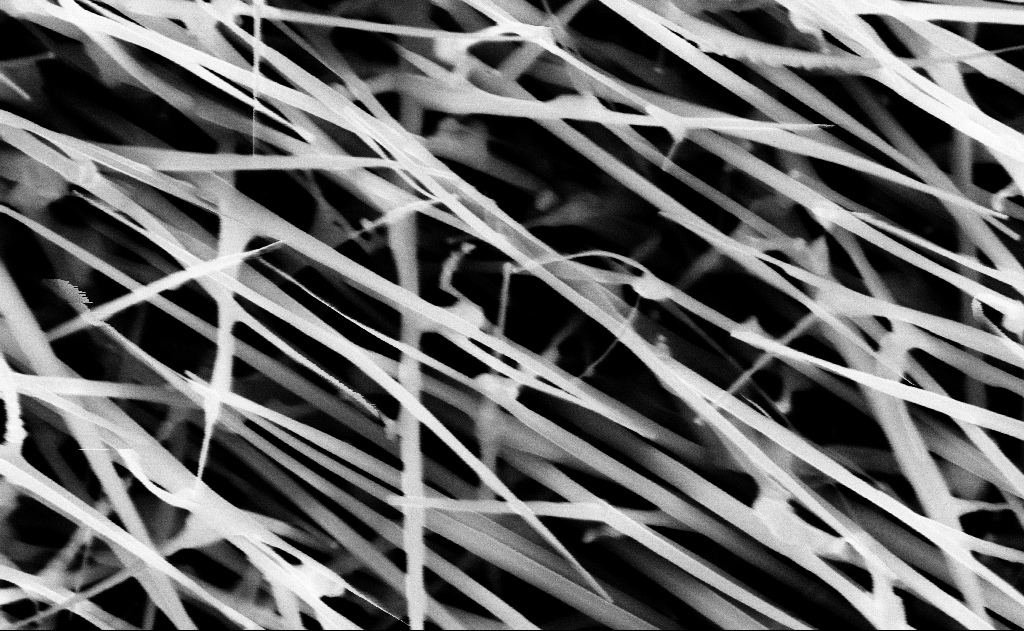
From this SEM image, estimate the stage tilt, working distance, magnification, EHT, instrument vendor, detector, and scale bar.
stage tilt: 0°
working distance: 13 mm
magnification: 80 K X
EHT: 10 kV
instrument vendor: Zeiss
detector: InLens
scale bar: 200 nm